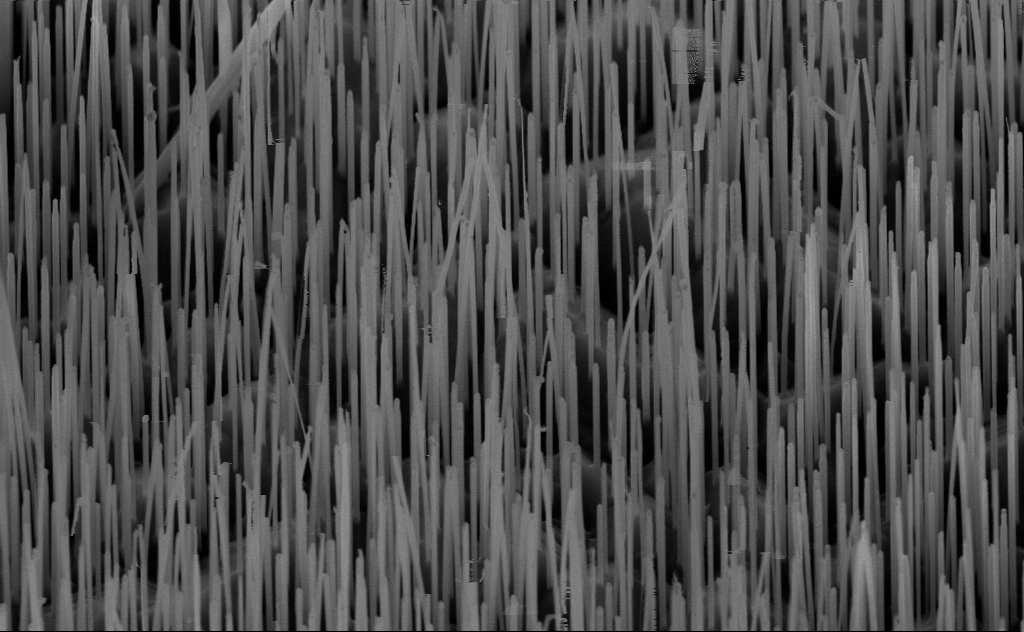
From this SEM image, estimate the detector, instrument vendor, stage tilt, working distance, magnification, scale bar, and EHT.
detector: InLens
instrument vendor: Zeiss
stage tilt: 45°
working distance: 6 mm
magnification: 40 K X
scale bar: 1000 nm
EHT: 10 kV